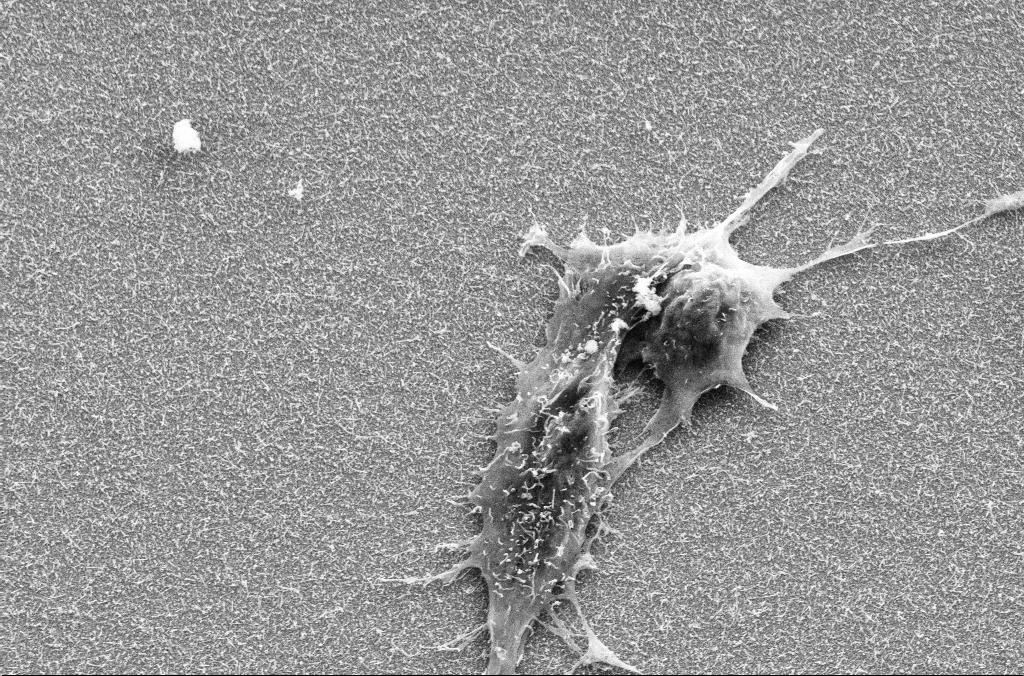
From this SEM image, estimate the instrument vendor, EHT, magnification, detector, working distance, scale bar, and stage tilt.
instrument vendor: Zeiss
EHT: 10 kV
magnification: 5.43 K X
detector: SE2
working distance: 8.1 mm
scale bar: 10000 nm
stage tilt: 17.3°